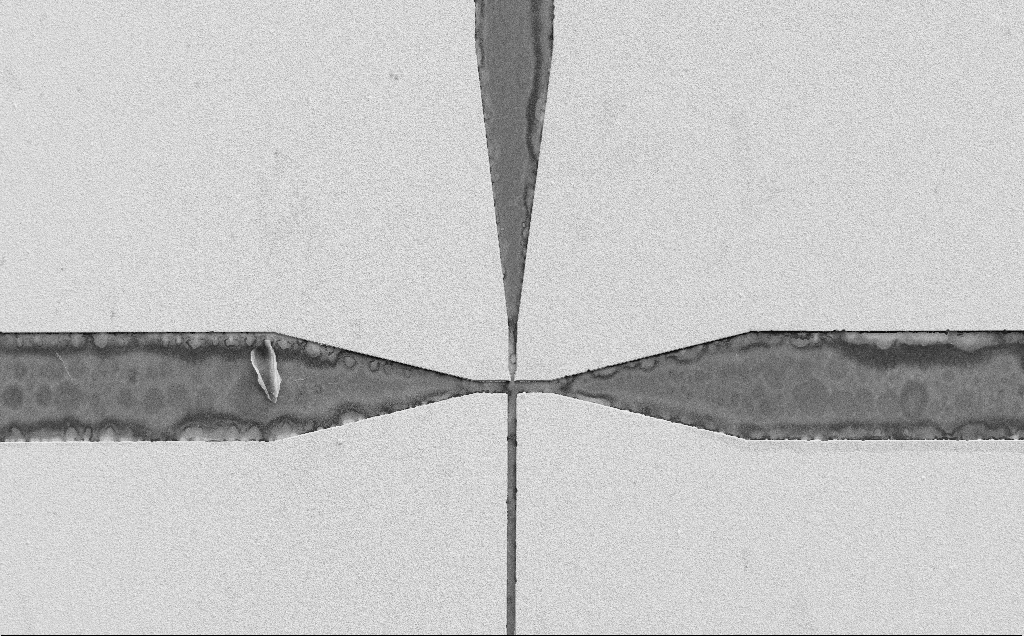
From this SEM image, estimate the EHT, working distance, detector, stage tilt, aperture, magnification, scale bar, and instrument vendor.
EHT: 10 kV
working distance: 14 mm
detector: SE2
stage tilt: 0°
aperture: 30 µm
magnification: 0.363 K X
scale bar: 100000 nm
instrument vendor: Zeiss